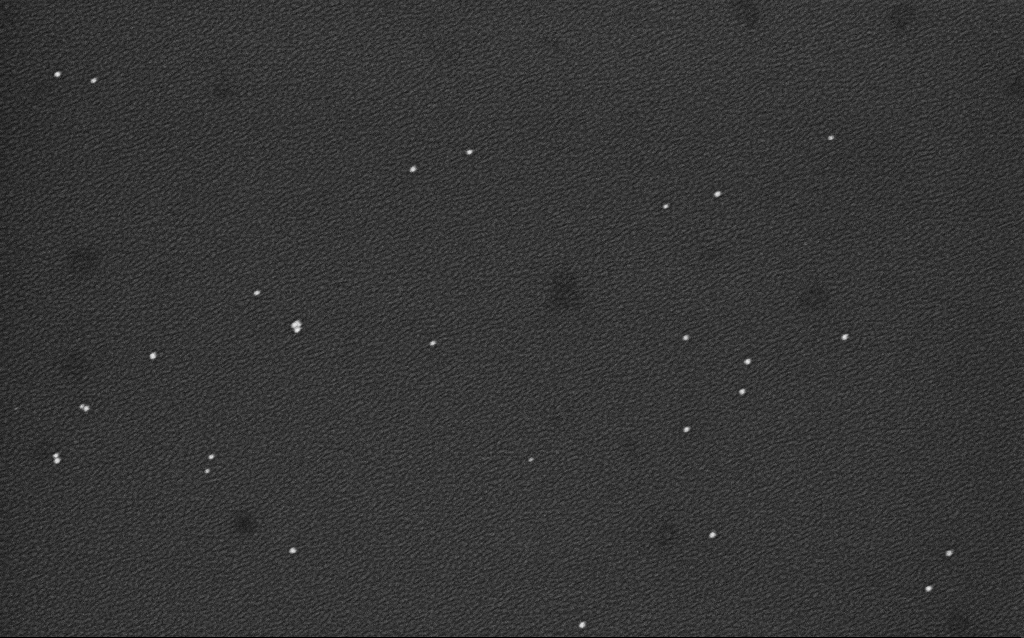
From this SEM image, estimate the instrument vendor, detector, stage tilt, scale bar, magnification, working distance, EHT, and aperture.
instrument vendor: Zeiss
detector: InLens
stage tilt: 0°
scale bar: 200 nm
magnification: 100 K X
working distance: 2.1 mm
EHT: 4 kV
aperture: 30 µm